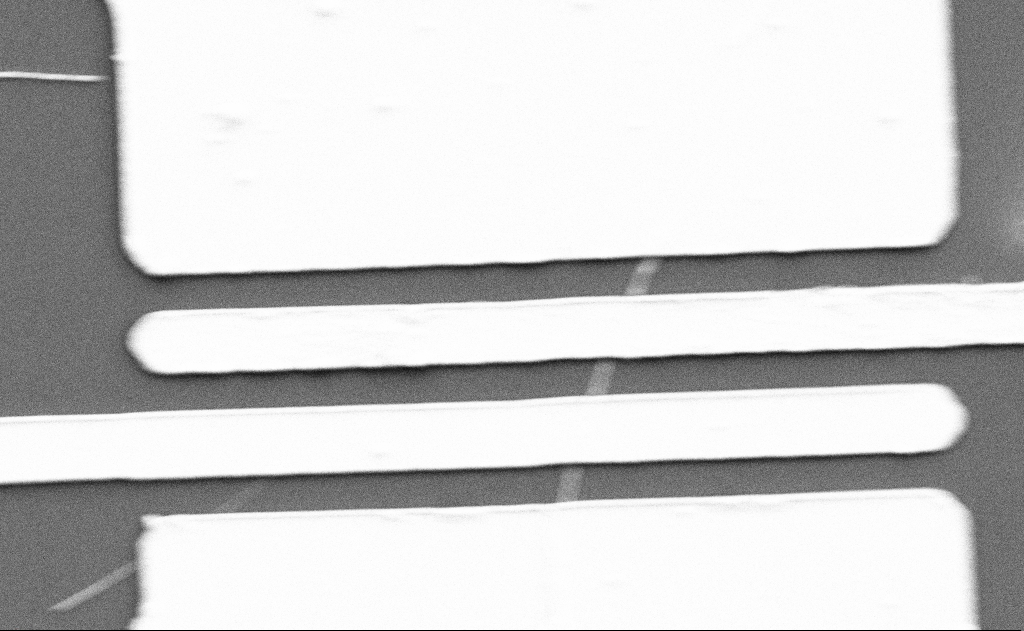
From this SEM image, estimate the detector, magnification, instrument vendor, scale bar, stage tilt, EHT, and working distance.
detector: SE2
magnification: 10 K X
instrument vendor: Zeiss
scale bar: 2000 nm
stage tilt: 0°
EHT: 5 kV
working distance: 15 mm